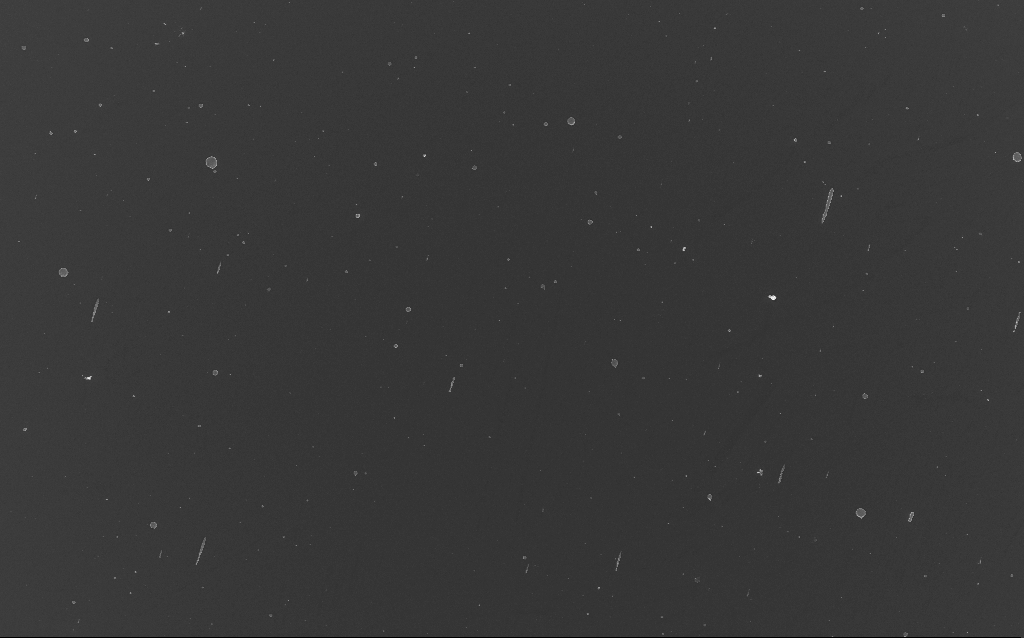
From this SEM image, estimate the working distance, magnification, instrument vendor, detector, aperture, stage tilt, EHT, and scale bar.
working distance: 4 mm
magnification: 1.23 K X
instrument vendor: Zeiss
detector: InLens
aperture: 30 µm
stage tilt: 0°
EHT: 10 kV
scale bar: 20000 nm